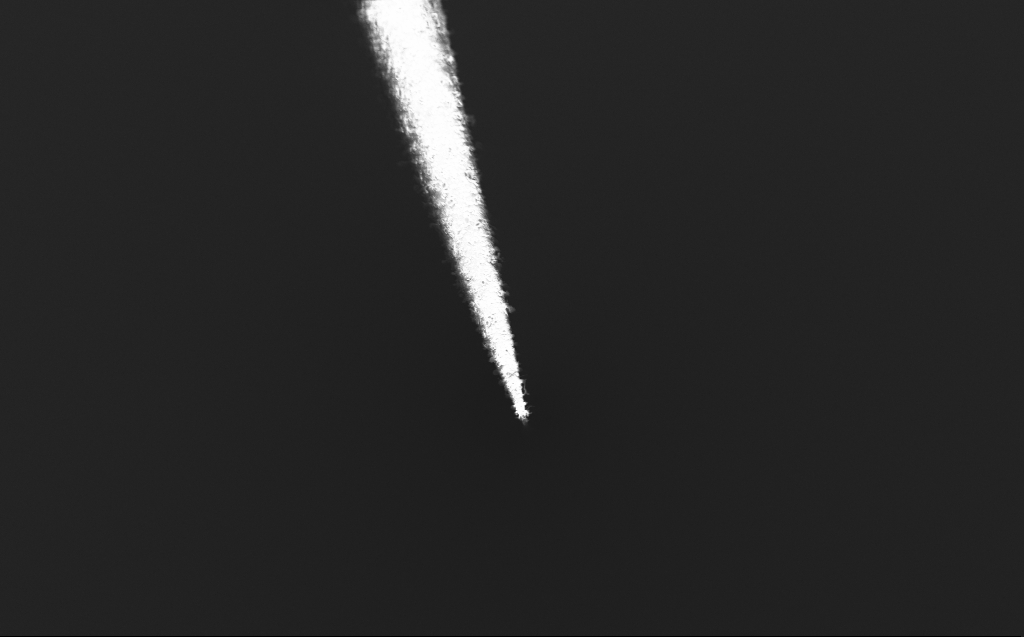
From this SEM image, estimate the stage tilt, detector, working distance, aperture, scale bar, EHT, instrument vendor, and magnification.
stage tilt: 45°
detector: InLens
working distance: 4 mm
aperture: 30 µm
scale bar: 2000 nm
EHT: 5 kV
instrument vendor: Zeiss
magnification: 7.34 K X